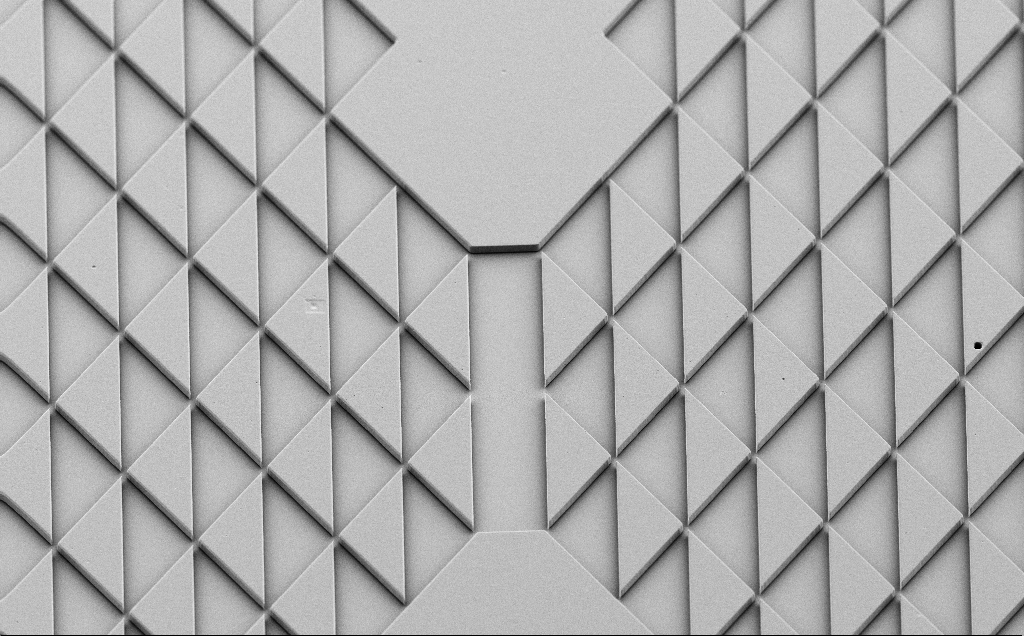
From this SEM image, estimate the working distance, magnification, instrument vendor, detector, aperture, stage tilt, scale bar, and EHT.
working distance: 9 mm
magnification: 0.73 K X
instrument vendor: Zeiss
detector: SE2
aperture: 30 µm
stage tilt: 40°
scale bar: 20000 nm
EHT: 5 kV